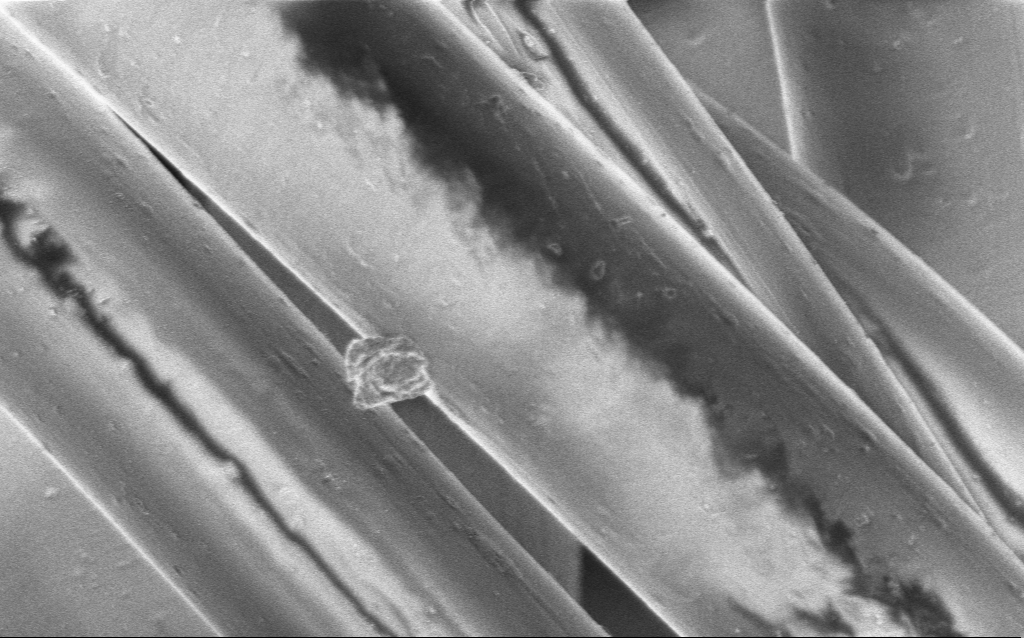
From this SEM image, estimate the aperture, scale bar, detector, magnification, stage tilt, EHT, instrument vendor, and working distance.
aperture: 30 µm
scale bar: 10000 nm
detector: InLens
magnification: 4.69 K X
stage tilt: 0°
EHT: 2 kV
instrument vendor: Zeiss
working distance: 5 mm